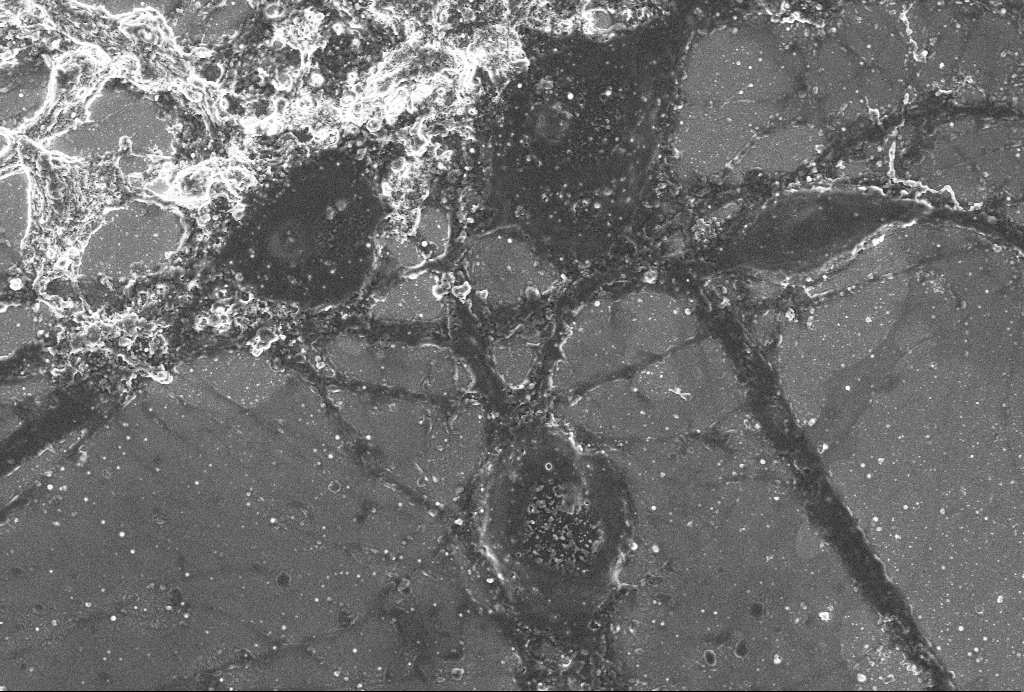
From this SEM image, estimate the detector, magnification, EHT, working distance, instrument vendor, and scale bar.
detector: SE2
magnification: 4 K X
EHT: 4 kV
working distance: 6 mm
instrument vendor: Zeiss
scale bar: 10000 nm